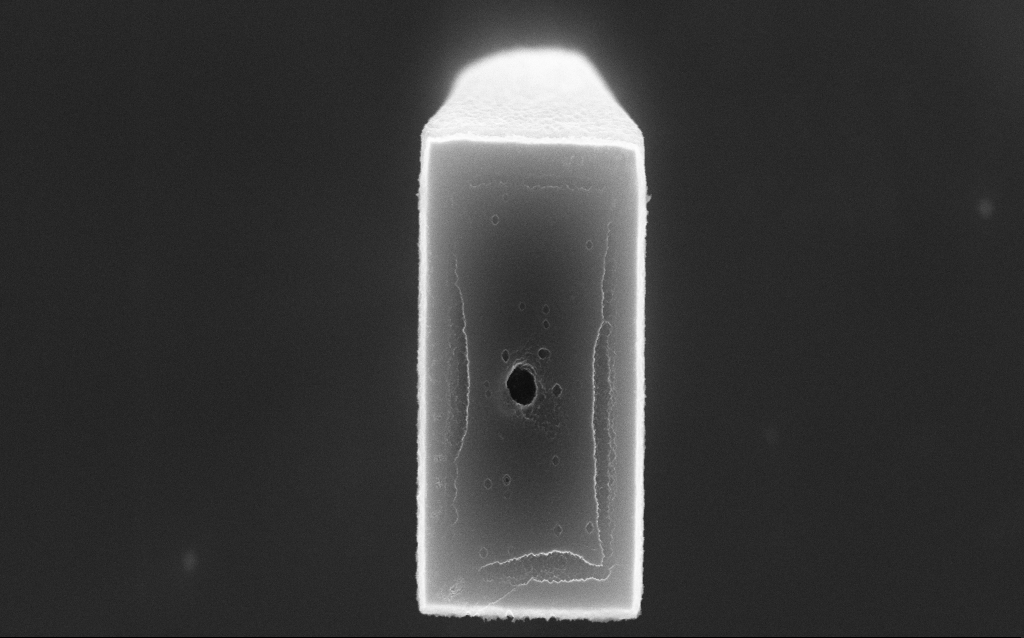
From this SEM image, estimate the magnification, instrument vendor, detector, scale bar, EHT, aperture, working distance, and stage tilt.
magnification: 45.23 K X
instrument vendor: Zeiss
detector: InLens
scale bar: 1000 nm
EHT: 10 kV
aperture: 30 µm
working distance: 8.3 mm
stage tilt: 0°